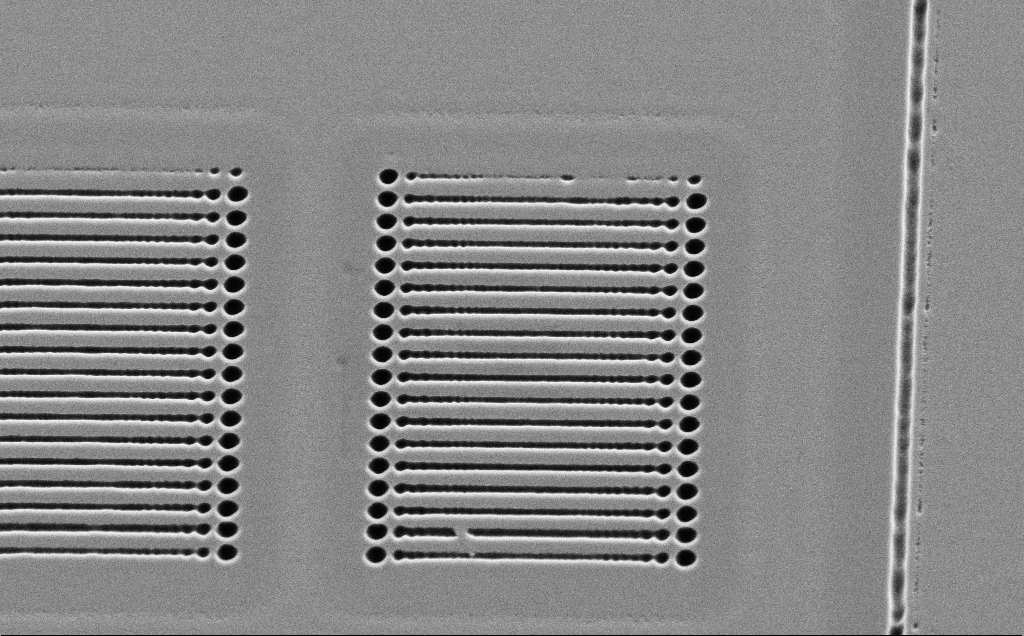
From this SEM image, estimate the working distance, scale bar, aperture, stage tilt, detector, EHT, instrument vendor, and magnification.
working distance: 10 mm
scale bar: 10000 nm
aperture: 30 µm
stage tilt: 45°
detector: SE2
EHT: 5 kV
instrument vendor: Zeiss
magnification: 4.18 K X